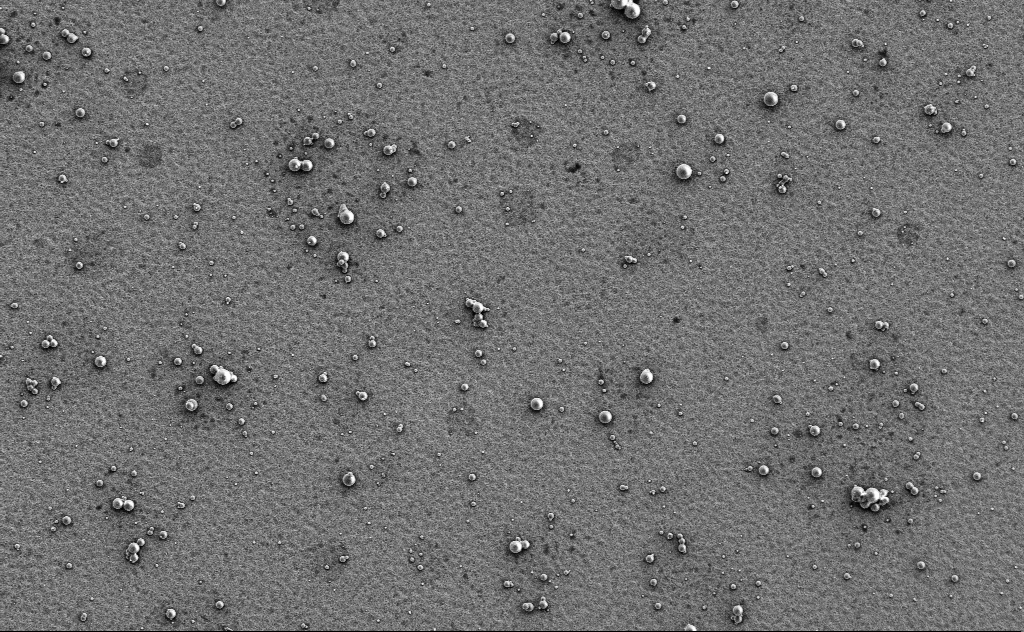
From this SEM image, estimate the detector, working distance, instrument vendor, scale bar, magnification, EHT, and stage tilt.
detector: SE2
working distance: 13 mm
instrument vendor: Zeiss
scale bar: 10000 nm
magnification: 2.61 K X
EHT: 3 kV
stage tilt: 0°